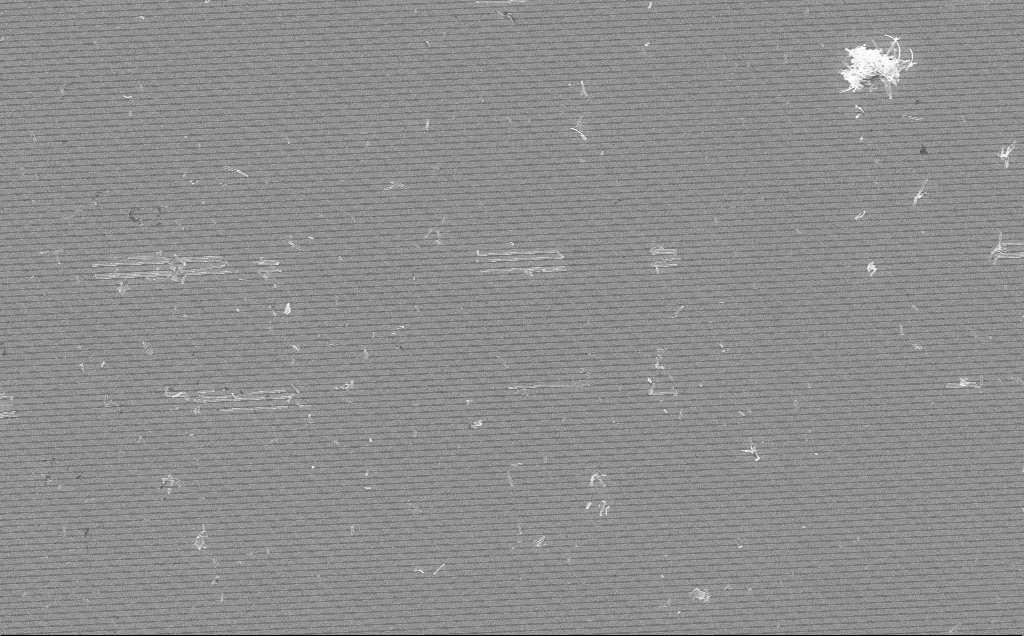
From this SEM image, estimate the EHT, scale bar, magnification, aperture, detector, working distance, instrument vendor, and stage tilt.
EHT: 10 kV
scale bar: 10000 nm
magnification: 5.9 K X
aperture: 30 µm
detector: InLens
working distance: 7 mm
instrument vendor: Zeiss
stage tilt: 0°